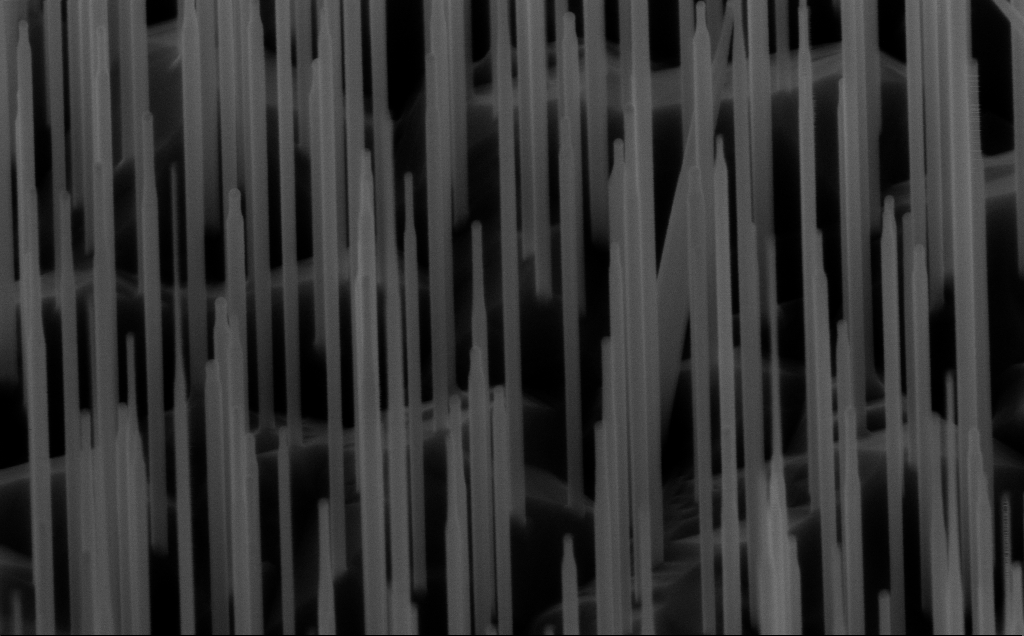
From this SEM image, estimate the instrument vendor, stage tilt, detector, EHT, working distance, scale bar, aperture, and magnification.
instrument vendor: Zeiss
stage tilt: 45°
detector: InLens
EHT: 10 kV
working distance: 6 mm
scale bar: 200 nm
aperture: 30 µm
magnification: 80 K X